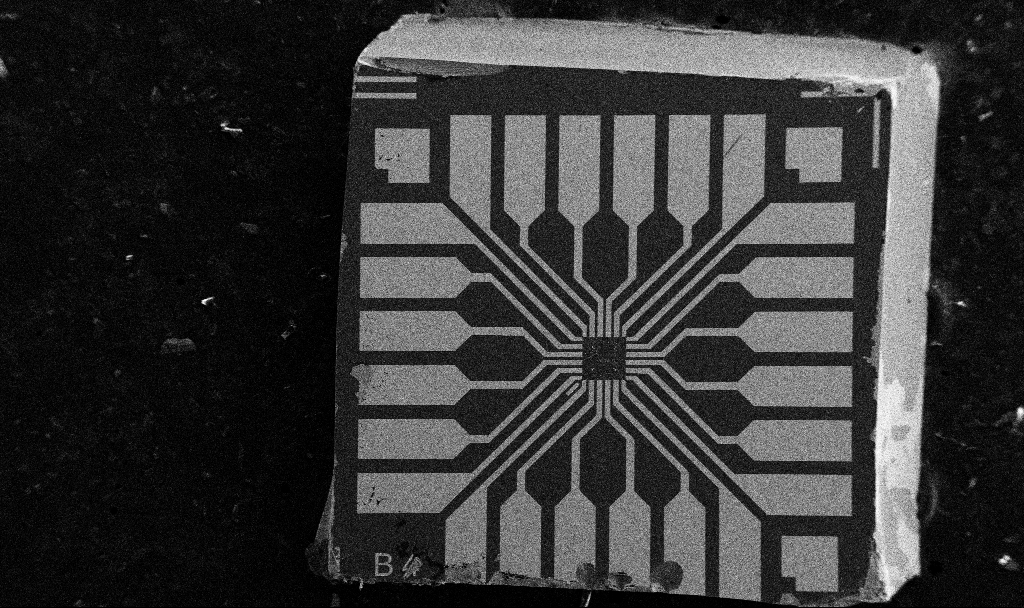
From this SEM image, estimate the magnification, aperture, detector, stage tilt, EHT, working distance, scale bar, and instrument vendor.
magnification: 0.1 K X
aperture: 30 µm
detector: SE2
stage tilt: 0°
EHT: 5 kV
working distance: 10.7 mm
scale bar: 200000 nm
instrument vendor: Zeiss